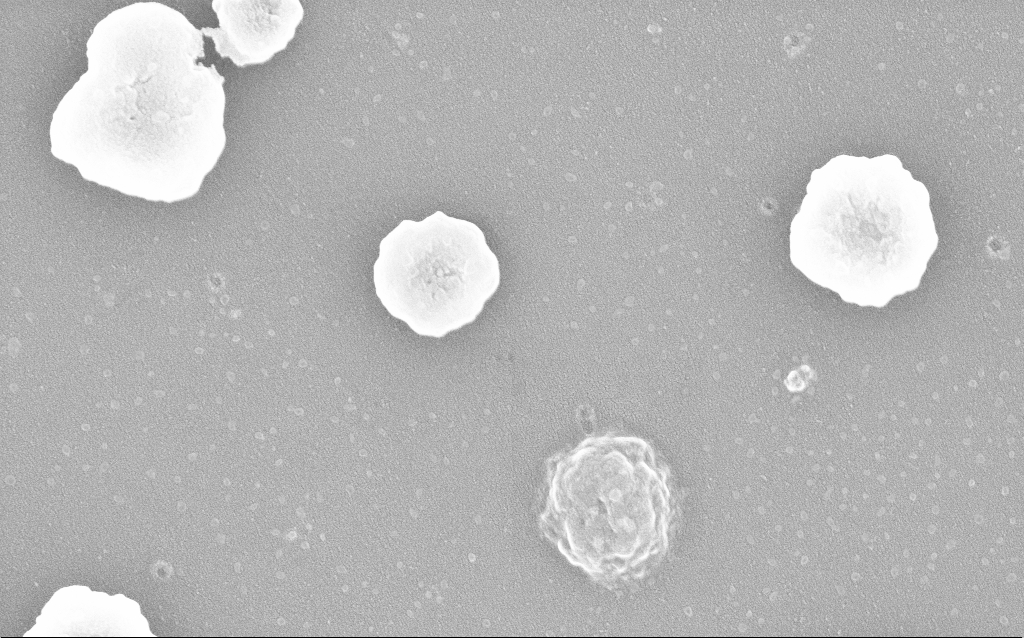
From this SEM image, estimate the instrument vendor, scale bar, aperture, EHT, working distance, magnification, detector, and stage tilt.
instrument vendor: Zeiss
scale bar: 200 nm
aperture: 30 µm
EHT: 20 kV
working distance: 1.4 mm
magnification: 100 K X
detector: InLens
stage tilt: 0°